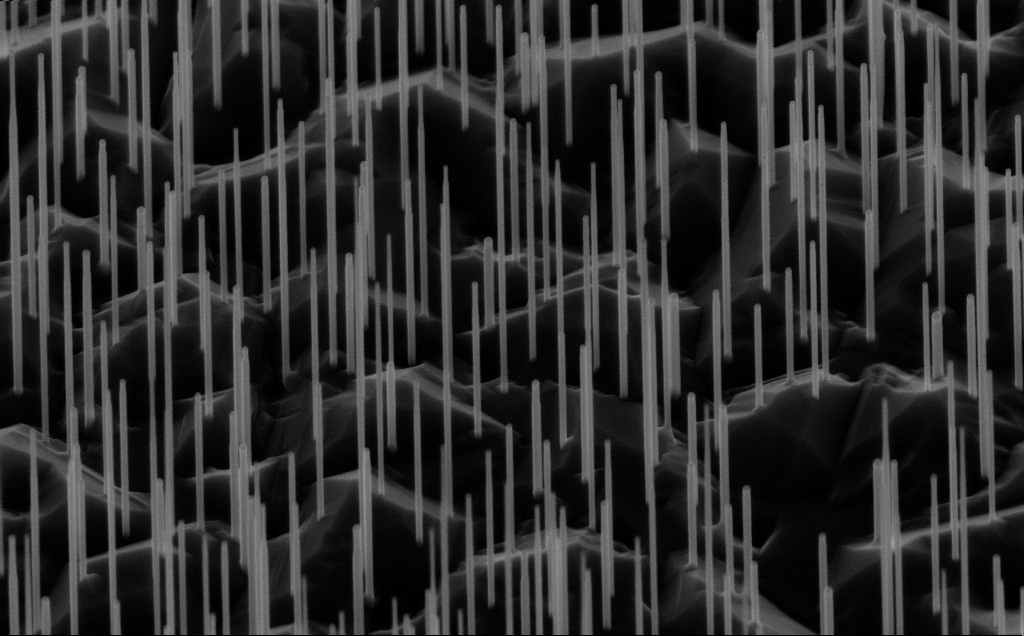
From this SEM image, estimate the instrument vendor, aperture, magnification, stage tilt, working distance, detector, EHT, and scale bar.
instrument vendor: Zeiss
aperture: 30 µm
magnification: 40 K X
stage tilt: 45°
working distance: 6 mm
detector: InLens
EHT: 10 kV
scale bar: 1000 nm